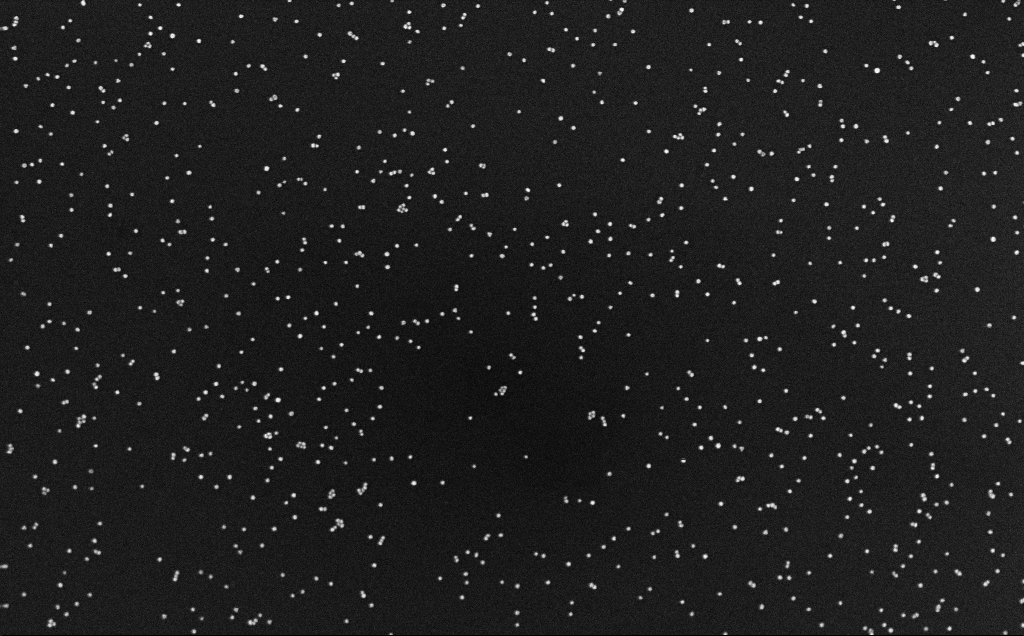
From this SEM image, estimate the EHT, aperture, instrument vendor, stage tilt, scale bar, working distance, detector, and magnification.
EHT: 10 kV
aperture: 30 µm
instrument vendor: Zeiss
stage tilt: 0°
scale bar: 200 nm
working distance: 3.1 mm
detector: InLens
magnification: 100 K X